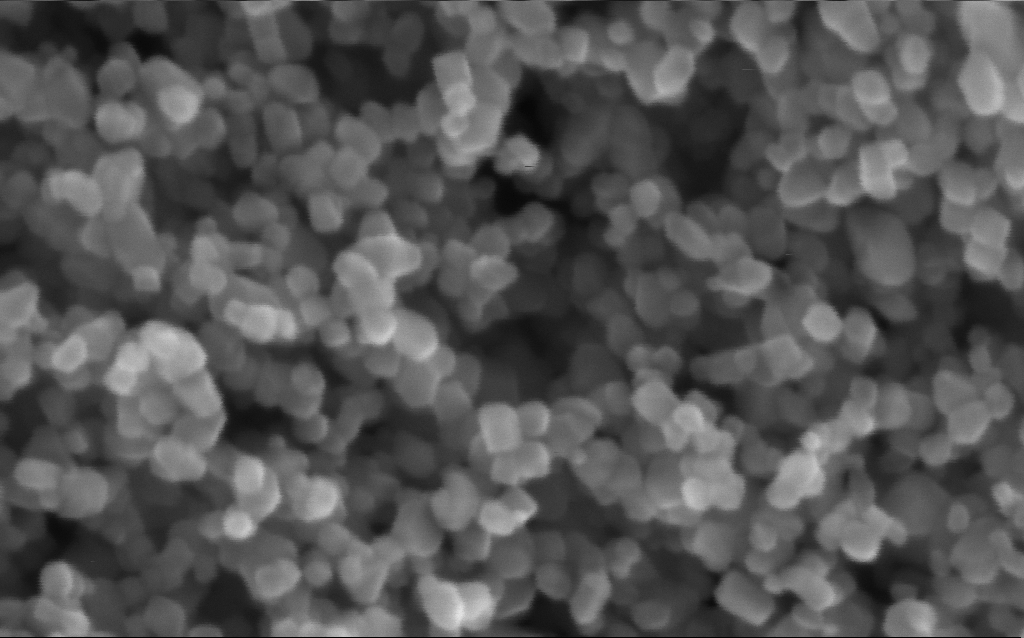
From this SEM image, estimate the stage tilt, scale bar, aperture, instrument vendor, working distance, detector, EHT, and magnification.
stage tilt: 0°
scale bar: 100 nm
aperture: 30 µm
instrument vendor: Zeiss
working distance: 3 mm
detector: InLens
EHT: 5 kV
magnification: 600 K X